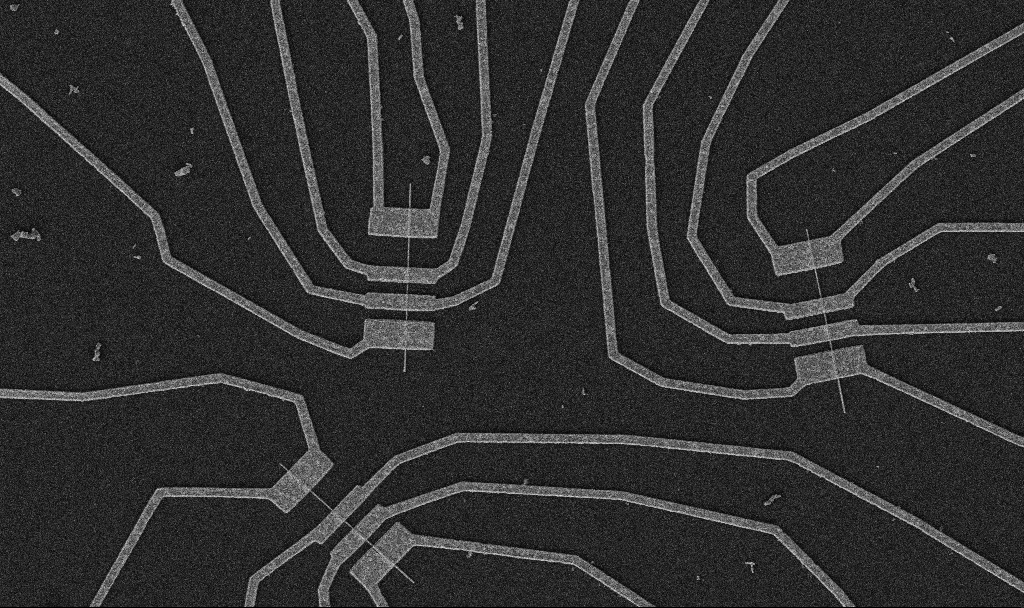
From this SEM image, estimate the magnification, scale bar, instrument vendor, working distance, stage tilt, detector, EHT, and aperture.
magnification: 5 K X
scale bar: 10000 nm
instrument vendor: Zeiss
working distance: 10.7 mm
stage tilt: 0°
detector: SE2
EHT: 5 kV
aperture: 30 µm